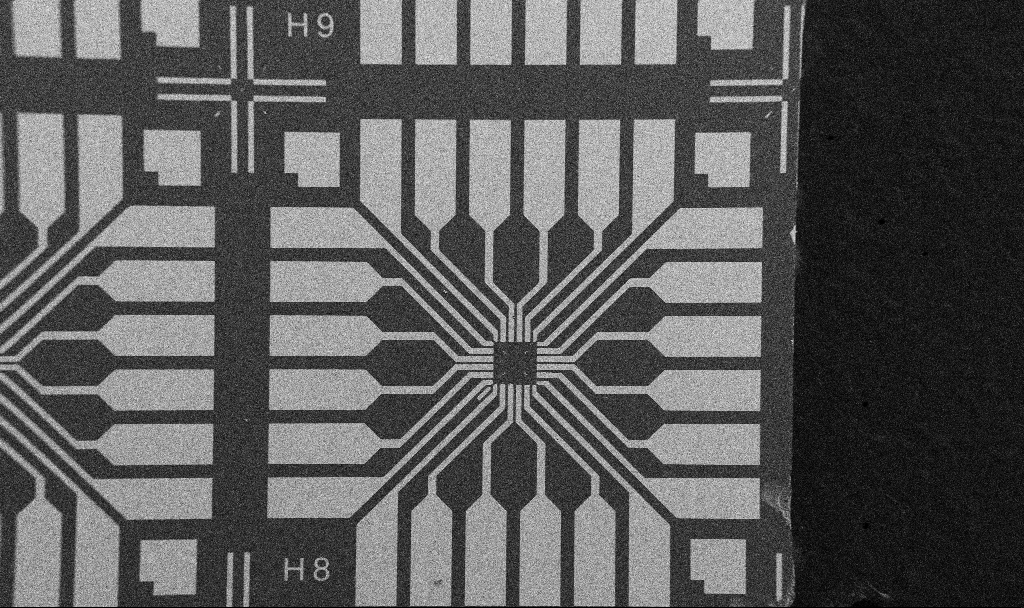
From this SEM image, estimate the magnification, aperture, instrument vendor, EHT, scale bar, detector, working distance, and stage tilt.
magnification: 0.1 K X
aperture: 30 µm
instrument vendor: Zeiss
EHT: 5 kV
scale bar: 200000 nm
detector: SE2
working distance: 10.7 mm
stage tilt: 0°